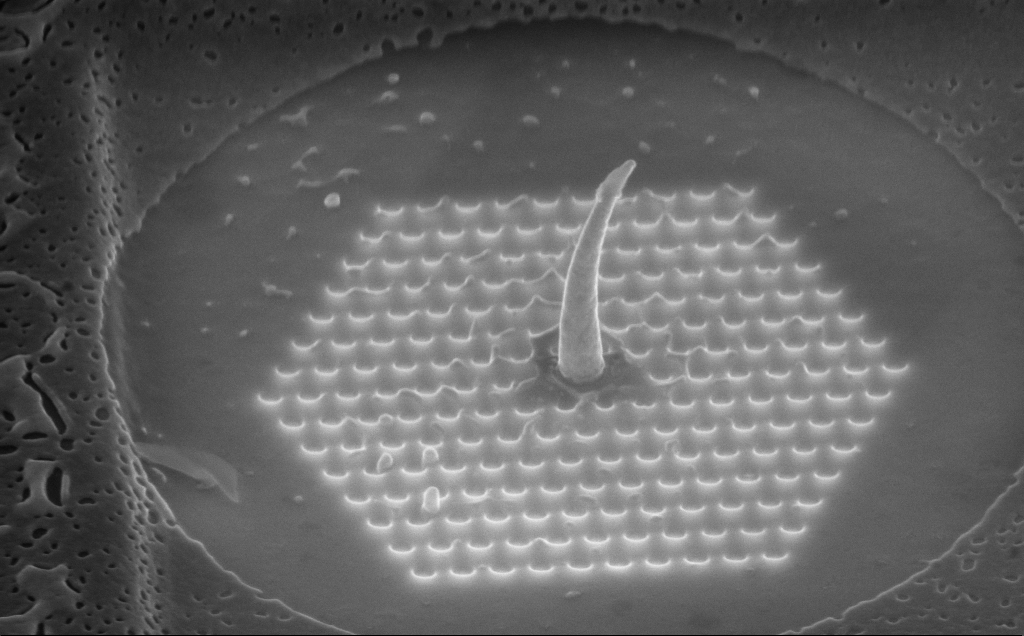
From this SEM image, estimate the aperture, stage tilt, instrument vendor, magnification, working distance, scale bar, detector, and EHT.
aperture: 30 µm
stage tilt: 45°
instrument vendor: Zeiss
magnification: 57.77 K X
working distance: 8 mm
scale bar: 1000 nm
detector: InLens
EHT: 10 kV